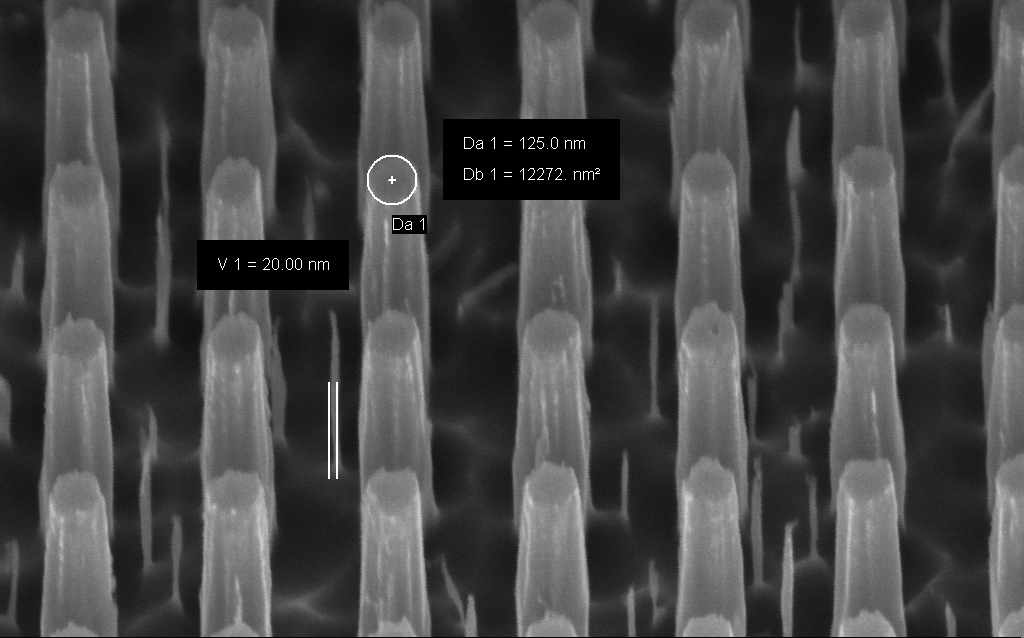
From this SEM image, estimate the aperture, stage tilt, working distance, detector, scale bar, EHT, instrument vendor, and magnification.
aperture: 30 µm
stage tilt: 45°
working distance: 5 mm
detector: InLens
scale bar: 200 nm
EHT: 3 kV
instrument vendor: Zeiss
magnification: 146.88 K X